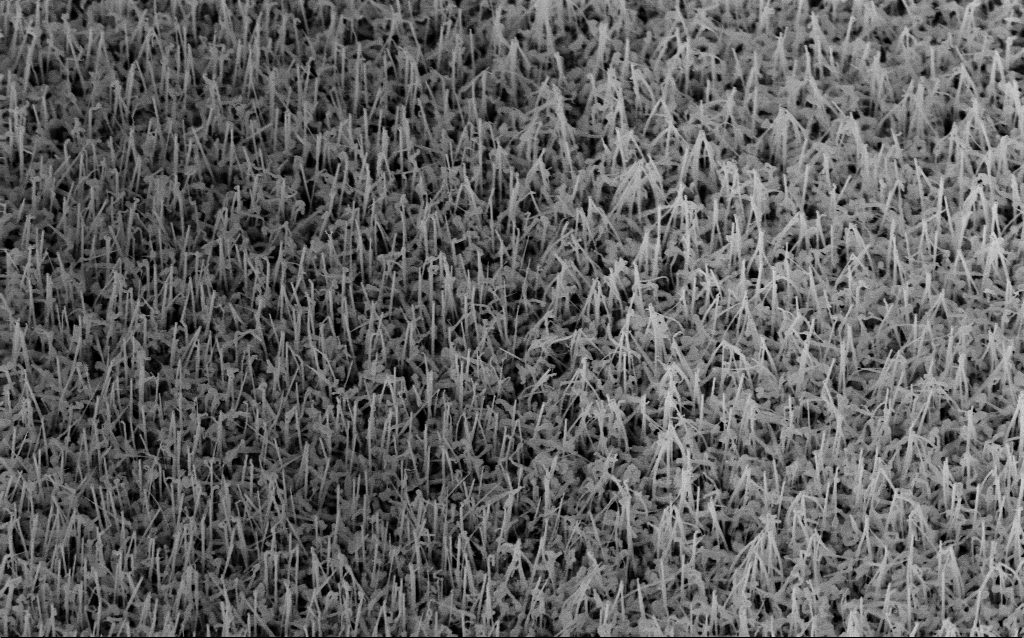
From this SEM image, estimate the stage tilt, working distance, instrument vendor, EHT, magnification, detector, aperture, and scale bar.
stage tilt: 35°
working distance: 7.3 mm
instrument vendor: Zeiss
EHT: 10 kV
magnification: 30 K X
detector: InLens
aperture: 30 µm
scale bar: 1000 nm